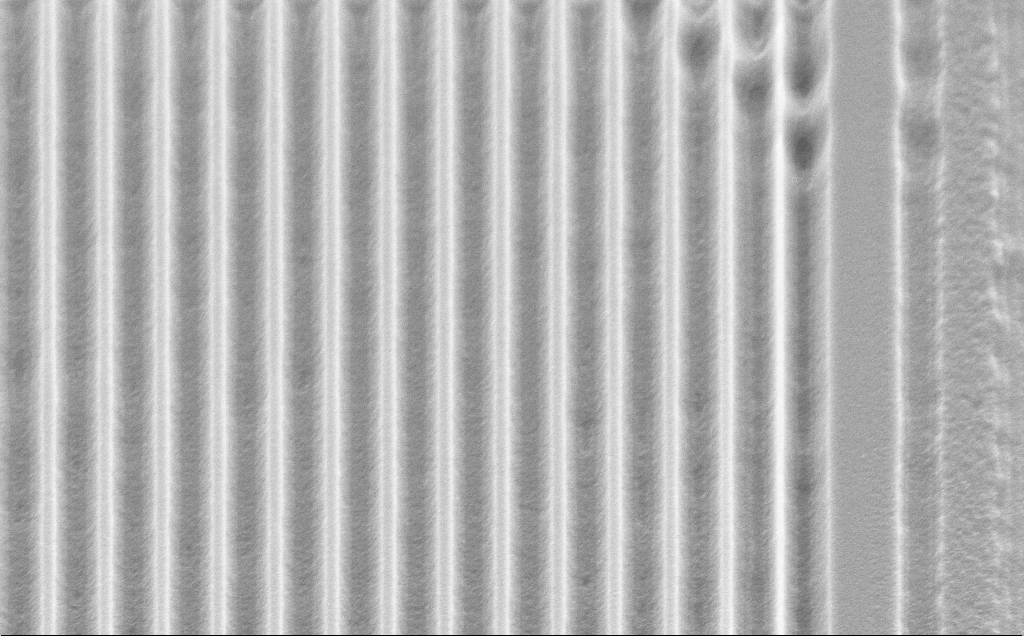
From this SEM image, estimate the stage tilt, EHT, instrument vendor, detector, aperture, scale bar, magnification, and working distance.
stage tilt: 45°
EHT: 5 kV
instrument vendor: Zeiss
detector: SE2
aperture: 30 µm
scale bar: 2000 nm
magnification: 10.36 K X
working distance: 10 mm